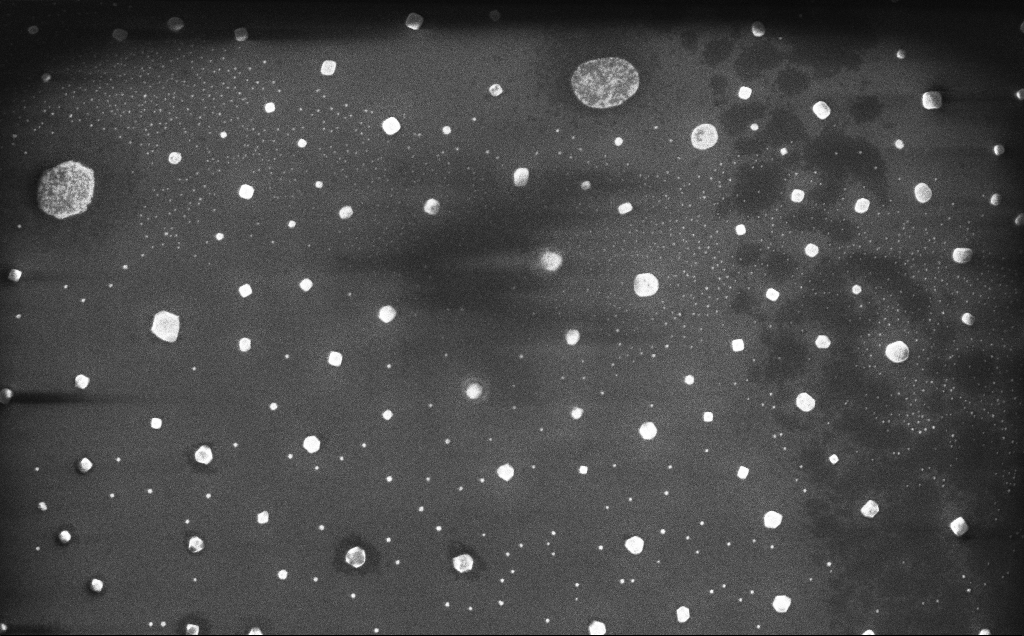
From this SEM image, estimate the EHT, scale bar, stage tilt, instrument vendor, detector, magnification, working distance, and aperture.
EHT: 10 kV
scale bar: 1000 nm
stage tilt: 0°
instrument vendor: Zeiss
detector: InLens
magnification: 21.66 K X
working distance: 5 mm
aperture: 30 µm